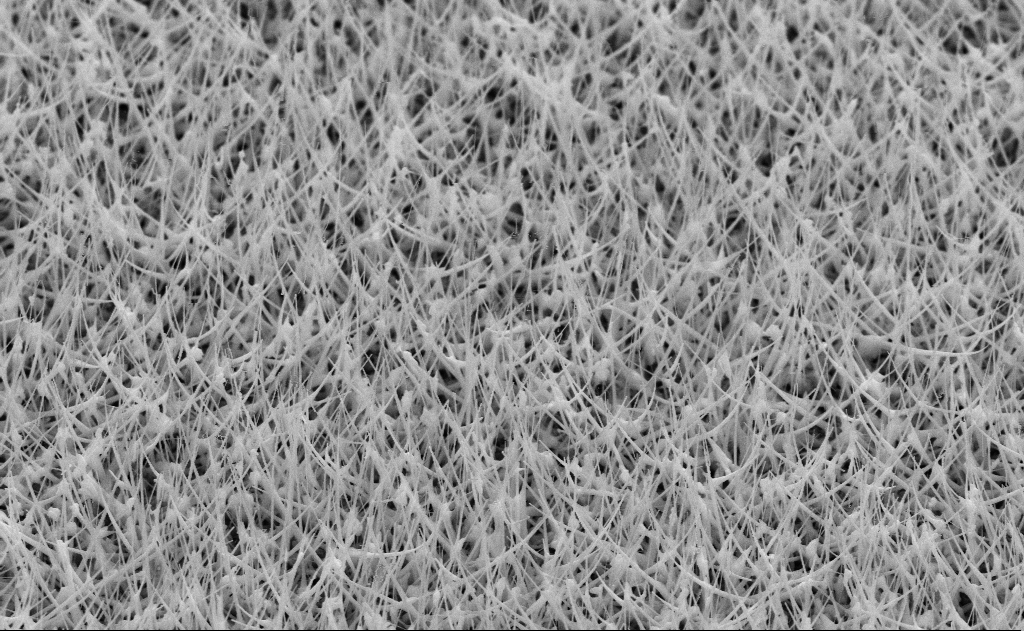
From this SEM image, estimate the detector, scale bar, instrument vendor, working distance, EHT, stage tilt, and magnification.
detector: SE2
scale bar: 2000 nm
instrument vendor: Zeiss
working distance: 11 mm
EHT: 10 kV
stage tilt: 45°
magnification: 20 K X